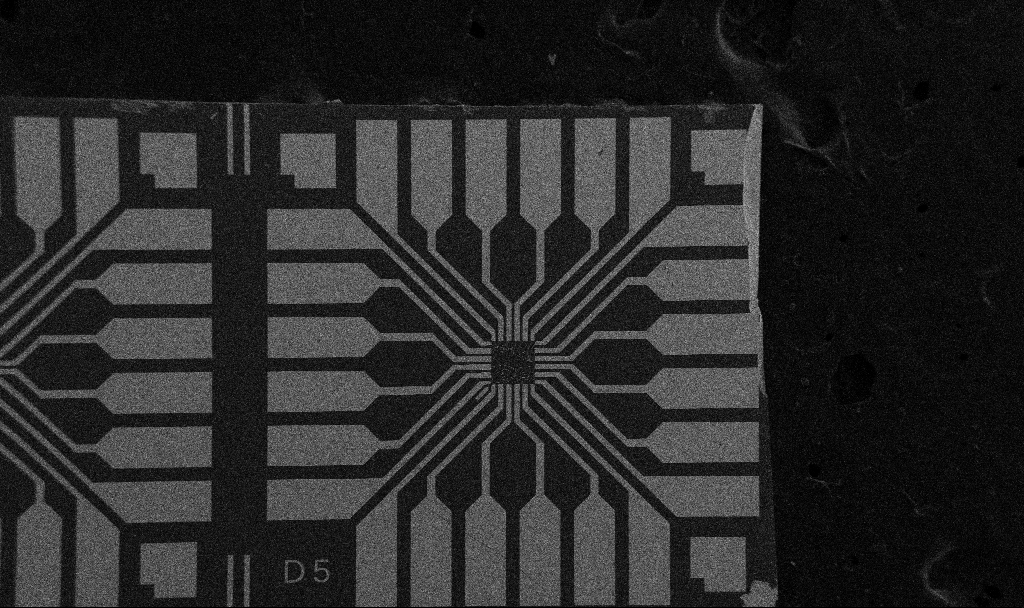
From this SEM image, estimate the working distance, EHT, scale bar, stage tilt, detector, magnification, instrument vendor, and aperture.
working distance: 10.7 mm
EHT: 5 kV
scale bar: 200000 nm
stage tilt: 0°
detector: SE2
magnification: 0.1 K X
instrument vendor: Zeiss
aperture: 30 µm